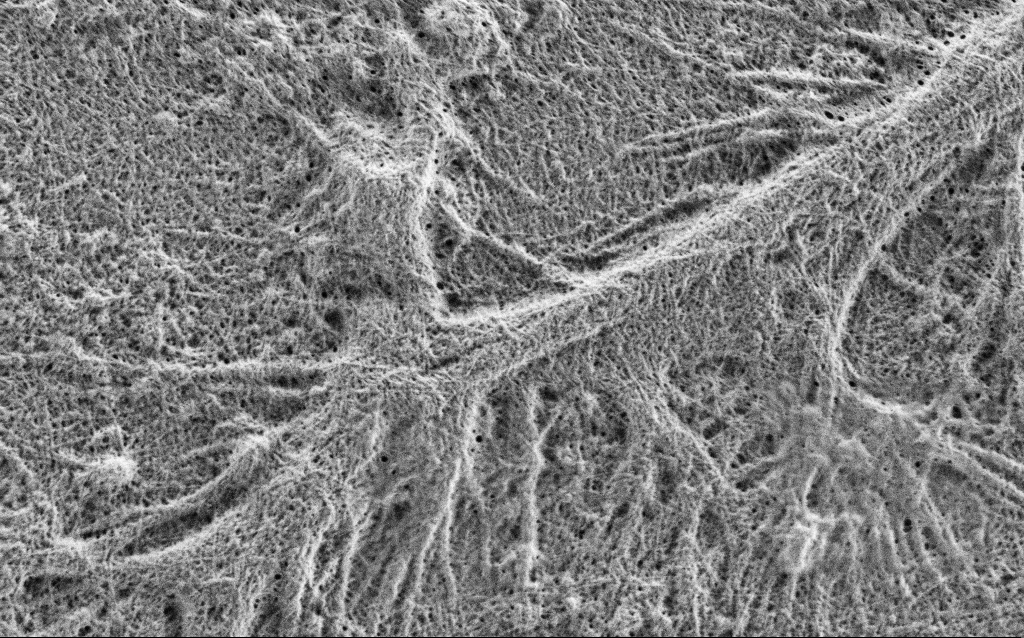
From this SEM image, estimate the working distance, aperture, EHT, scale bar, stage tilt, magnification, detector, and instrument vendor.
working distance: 4 mm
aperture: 30 µm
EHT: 0.9 kV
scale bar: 1000 nm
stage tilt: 0°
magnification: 20 K X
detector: SE2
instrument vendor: Zeiss